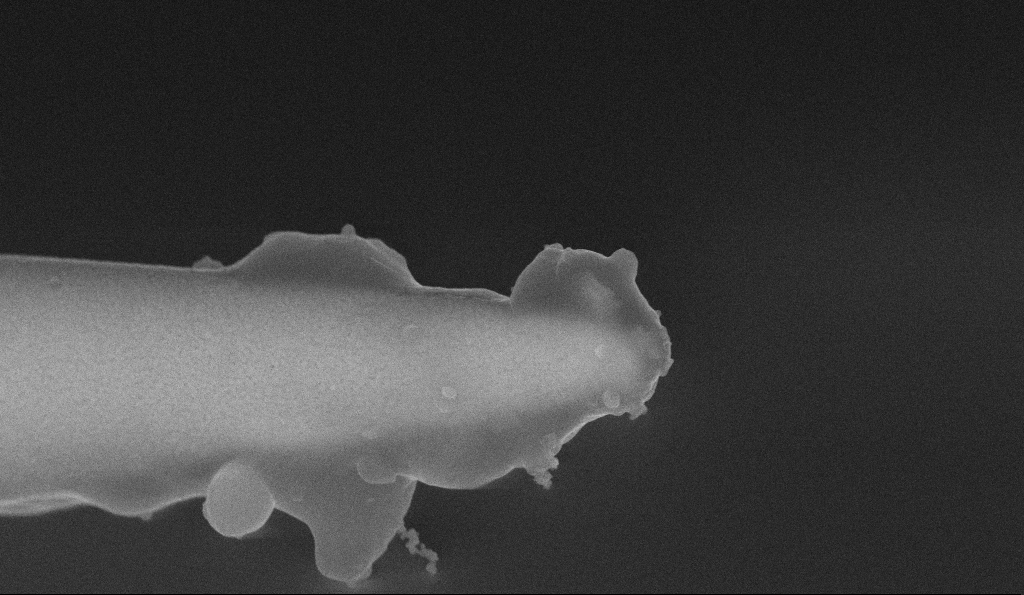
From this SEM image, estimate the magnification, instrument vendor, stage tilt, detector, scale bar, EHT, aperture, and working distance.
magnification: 50 K X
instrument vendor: Zeiss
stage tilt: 36.9°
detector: InLens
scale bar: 1000 nm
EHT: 10 kV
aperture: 30 µm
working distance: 4.1 mm